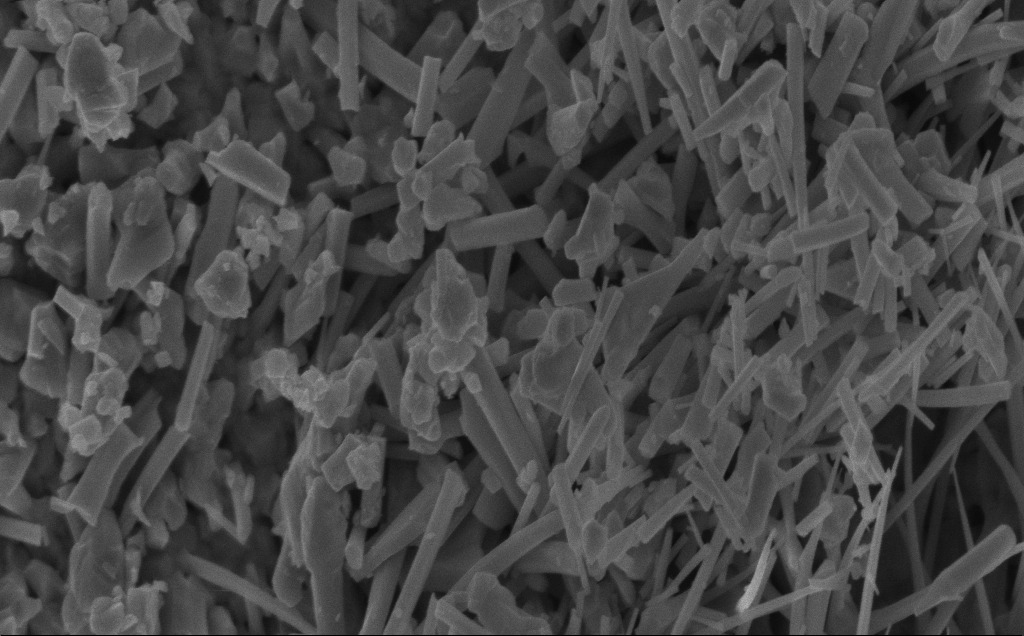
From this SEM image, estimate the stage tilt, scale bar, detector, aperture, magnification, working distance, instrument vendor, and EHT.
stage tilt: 0°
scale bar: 1000 nm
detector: InLens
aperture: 30 µm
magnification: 50.36 K X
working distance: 5 mm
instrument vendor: Zeiss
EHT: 5 kV